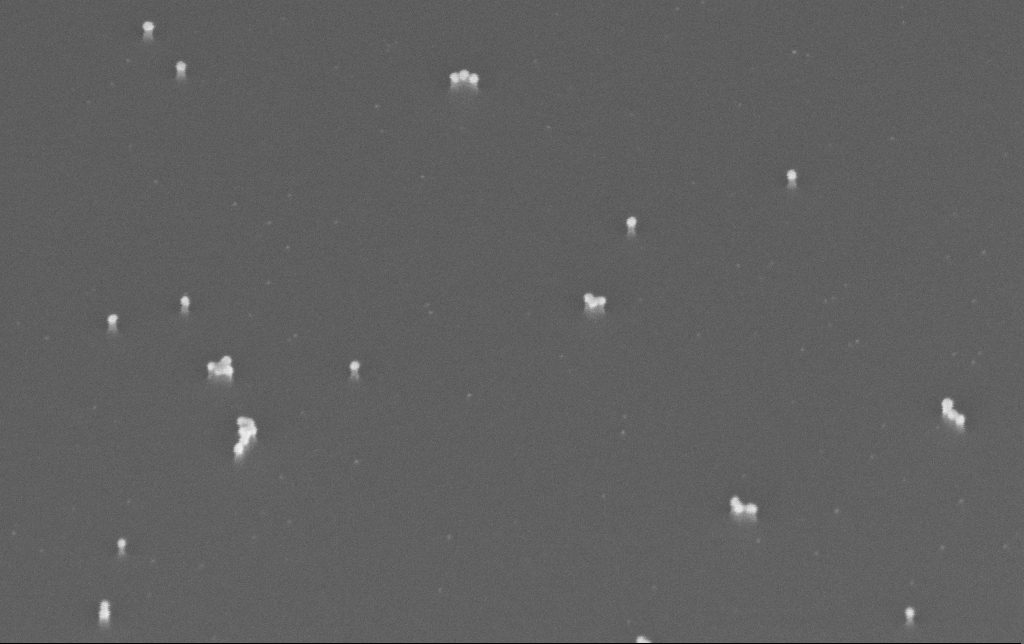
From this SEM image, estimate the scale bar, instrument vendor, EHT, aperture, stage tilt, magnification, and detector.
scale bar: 200 nm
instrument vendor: Zeiss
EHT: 10 kV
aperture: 30 µm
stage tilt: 45°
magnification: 200 K X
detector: InLens